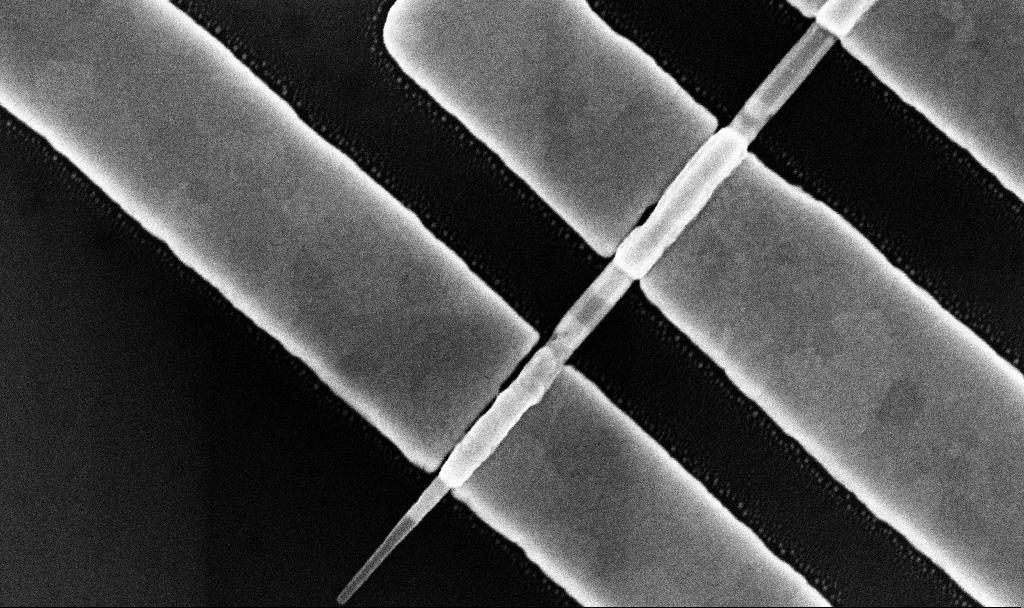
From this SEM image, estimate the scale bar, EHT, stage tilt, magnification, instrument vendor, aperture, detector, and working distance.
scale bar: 200 nm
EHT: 10 kV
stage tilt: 0°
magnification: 101.83 K X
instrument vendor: Zeiss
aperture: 30 µm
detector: InLens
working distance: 7.7 mm